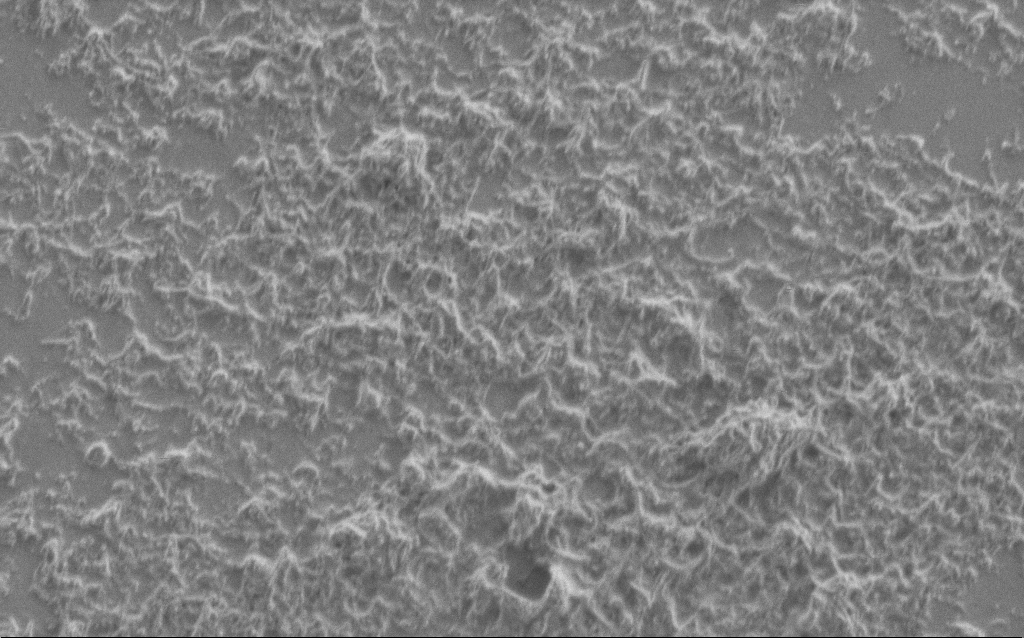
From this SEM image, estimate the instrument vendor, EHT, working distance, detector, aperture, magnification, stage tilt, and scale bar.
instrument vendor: Zeiss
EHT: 1 kV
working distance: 7 mm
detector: SE2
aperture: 30 µm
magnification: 25 K X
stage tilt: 0°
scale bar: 1000 nm